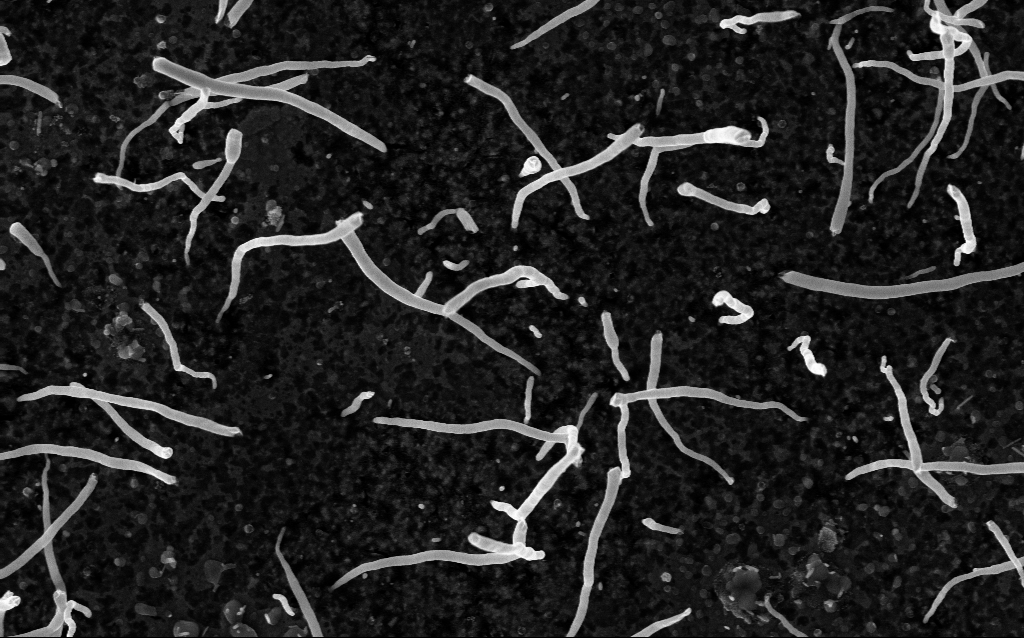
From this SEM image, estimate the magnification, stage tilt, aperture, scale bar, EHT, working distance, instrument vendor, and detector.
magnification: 50 K X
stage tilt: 0°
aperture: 30 µm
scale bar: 1000 nm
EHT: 5 kV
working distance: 2 mm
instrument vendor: Zeiss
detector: InLens